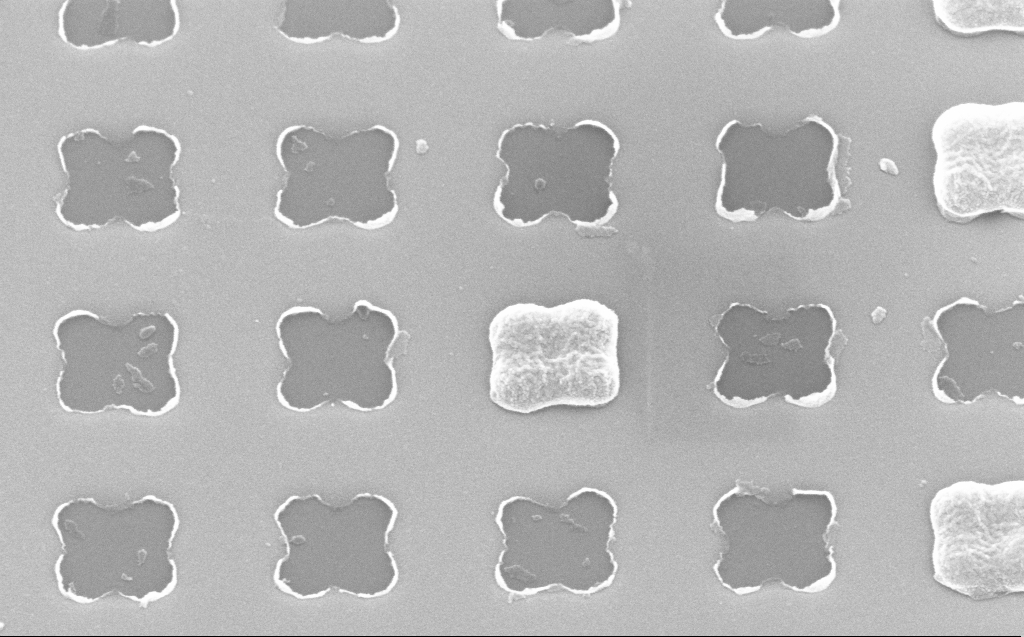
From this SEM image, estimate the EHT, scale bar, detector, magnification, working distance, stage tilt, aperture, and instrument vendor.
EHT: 10 kV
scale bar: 200 nm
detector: InLens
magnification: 162.44 K X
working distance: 5 mm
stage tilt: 30.9°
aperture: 30 µm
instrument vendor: Zeiss